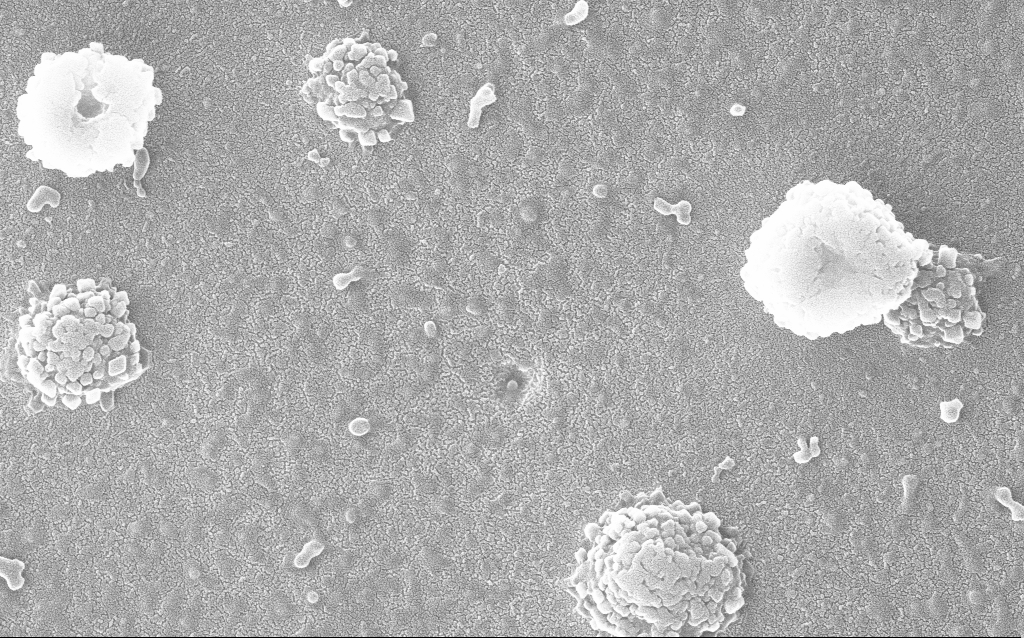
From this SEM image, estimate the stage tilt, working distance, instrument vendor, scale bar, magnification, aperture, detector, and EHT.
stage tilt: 0°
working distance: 1.5 mm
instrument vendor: Zeiss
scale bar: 200 nm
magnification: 100 K X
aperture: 30 µm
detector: InLens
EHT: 20 kV